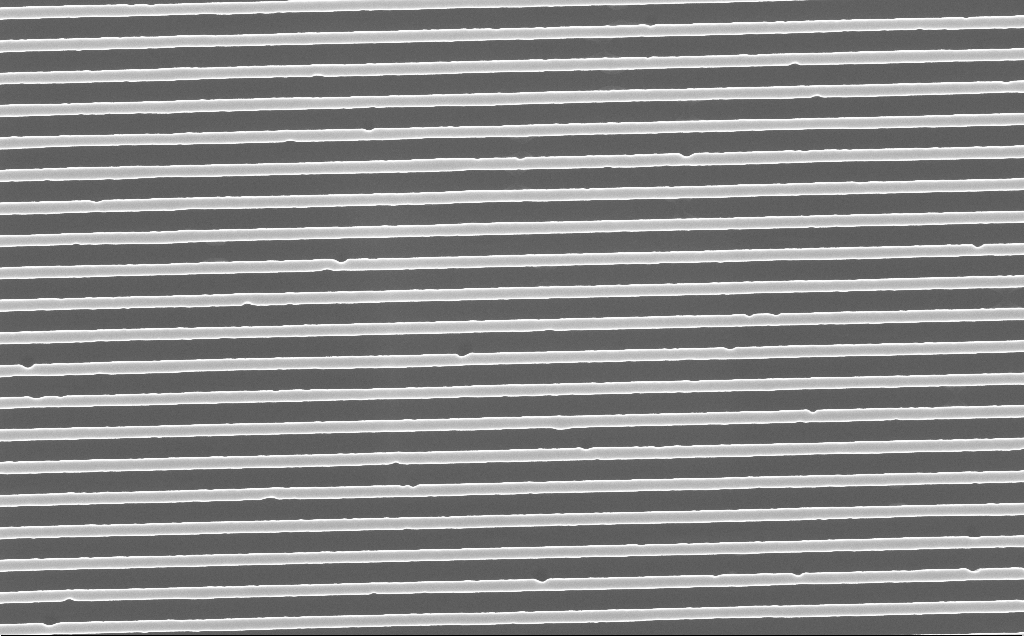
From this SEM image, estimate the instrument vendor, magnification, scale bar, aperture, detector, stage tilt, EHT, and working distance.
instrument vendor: Zeiss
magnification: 40 K X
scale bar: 1000 nm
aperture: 30 µm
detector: InLens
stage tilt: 0°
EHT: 10 kV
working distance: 4 mm